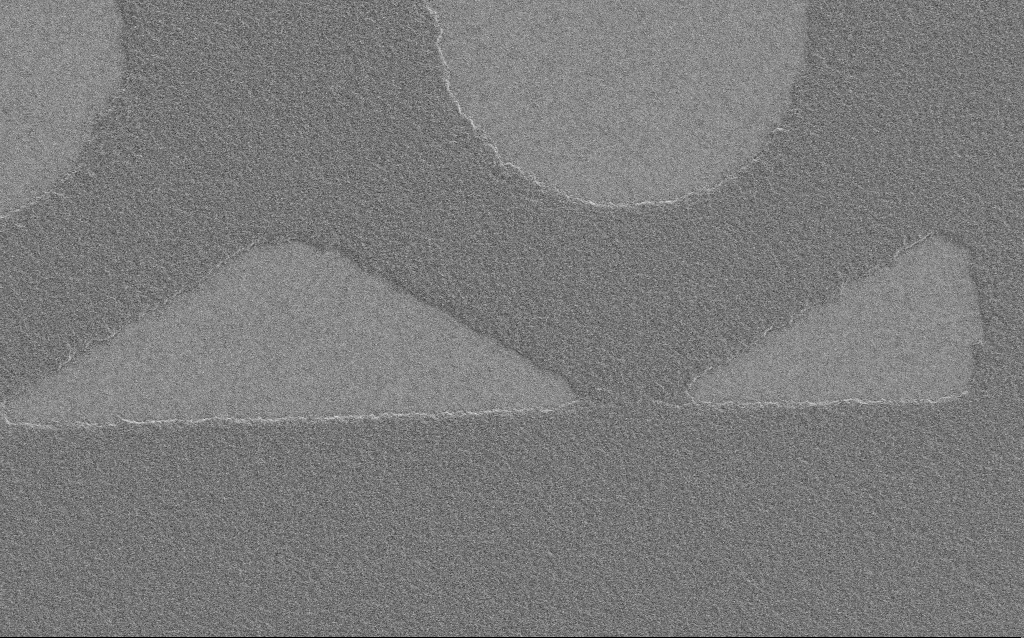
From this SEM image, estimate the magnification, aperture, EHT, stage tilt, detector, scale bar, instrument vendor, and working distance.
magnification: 3.68 K X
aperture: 30 µm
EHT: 2 kV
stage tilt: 0°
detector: SE2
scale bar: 10000 nm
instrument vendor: Zeiss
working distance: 4 mm